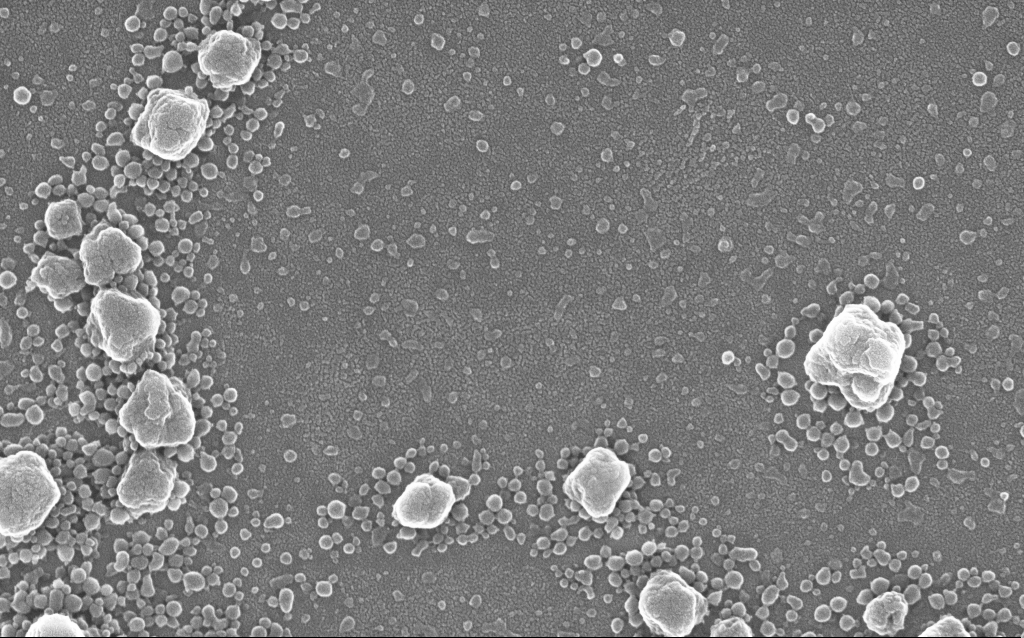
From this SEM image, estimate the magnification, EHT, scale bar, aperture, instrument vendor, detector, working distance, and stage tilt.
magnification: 100 K X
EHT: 20 kV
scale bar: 200 nm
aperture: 30 µm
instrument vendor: Zeiss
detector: InLens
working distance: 1.5 mm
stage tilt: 0°